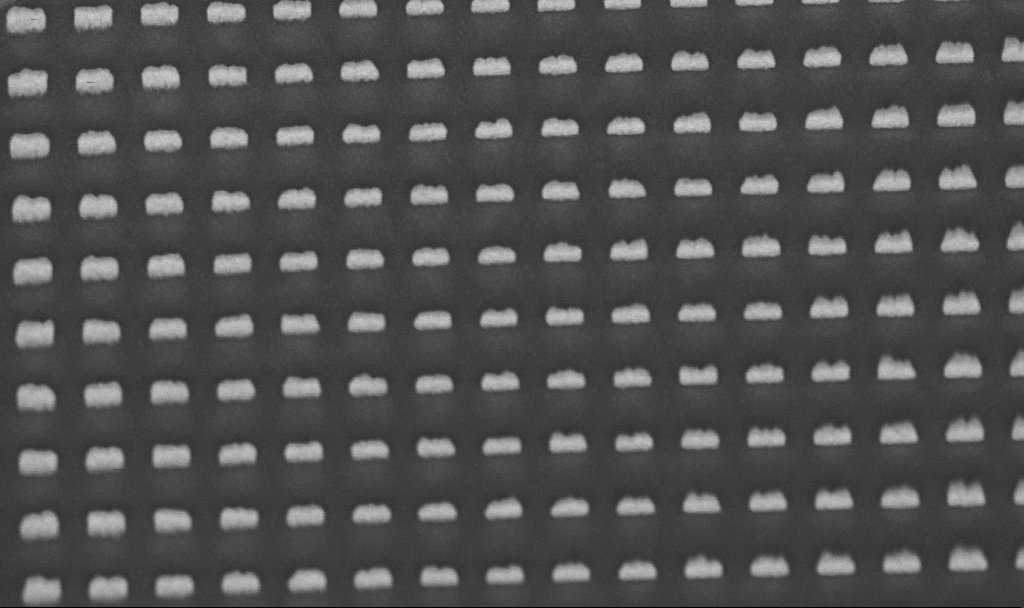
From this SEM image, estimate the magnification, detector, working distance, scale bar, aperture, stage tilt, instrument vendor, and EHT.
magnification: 105.49 K X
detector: InLens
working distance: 6.7 mm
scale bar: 200 nm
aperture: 30 µm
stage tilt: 45°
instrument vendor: Zeiss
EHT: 5 kV